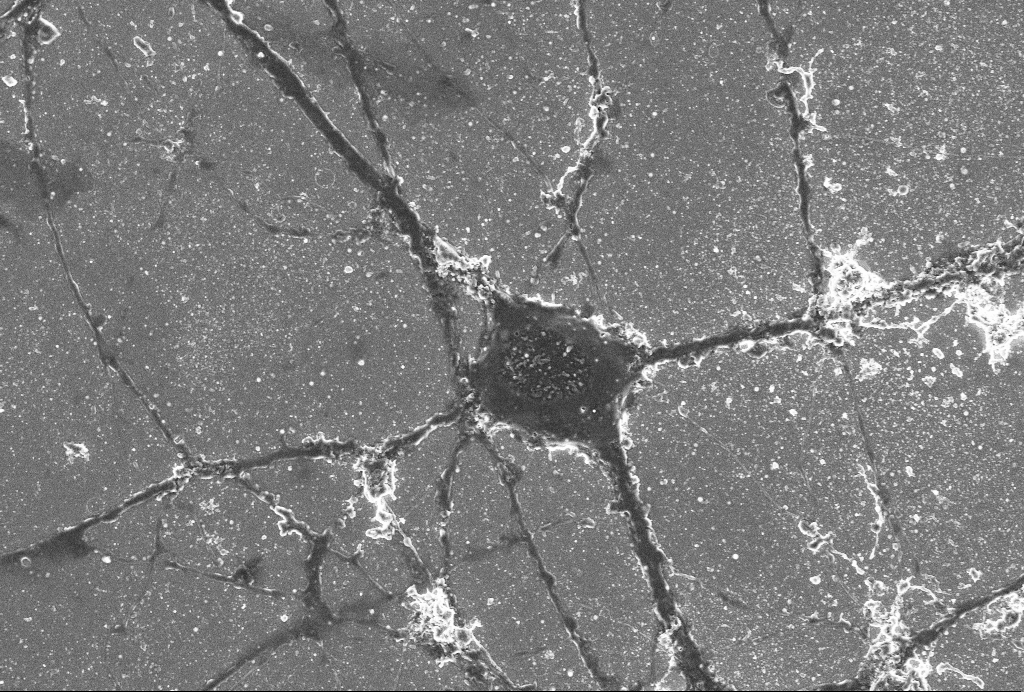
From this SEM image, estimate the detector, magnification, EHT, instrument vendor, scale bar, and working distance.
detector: SE2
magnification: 4 K X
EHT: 4 kV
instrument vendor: Zeiss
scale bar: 10000 nm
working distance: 6 mm